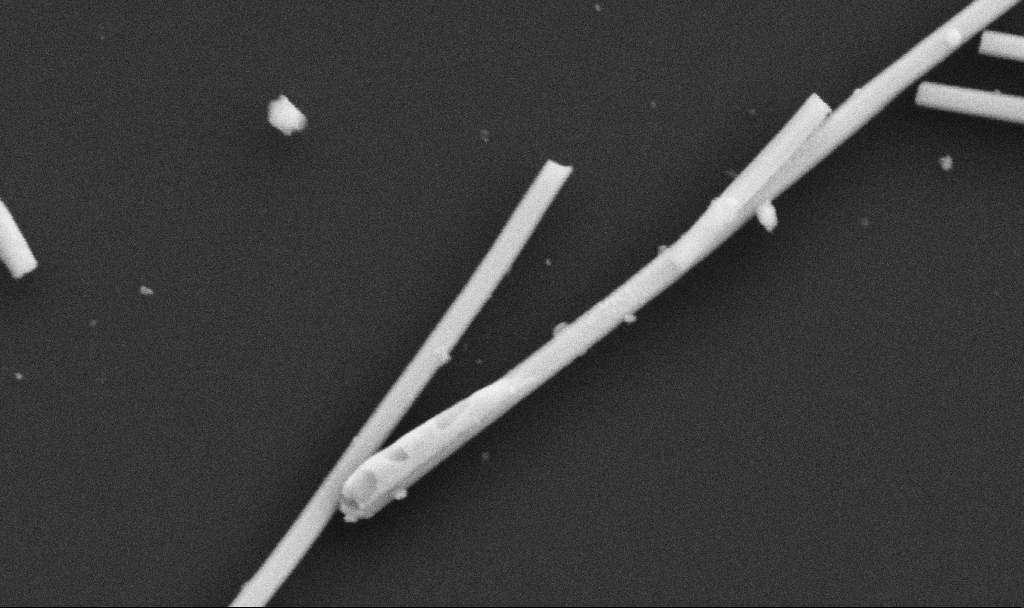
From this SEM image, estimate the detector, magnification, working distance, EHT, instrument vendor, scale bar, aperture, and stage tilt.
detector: SE2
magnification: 100.56 K X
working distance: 10.7 mm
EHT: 5 kV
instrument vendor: Zeiss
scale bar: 200 nm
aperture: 30 µm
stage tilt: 0°